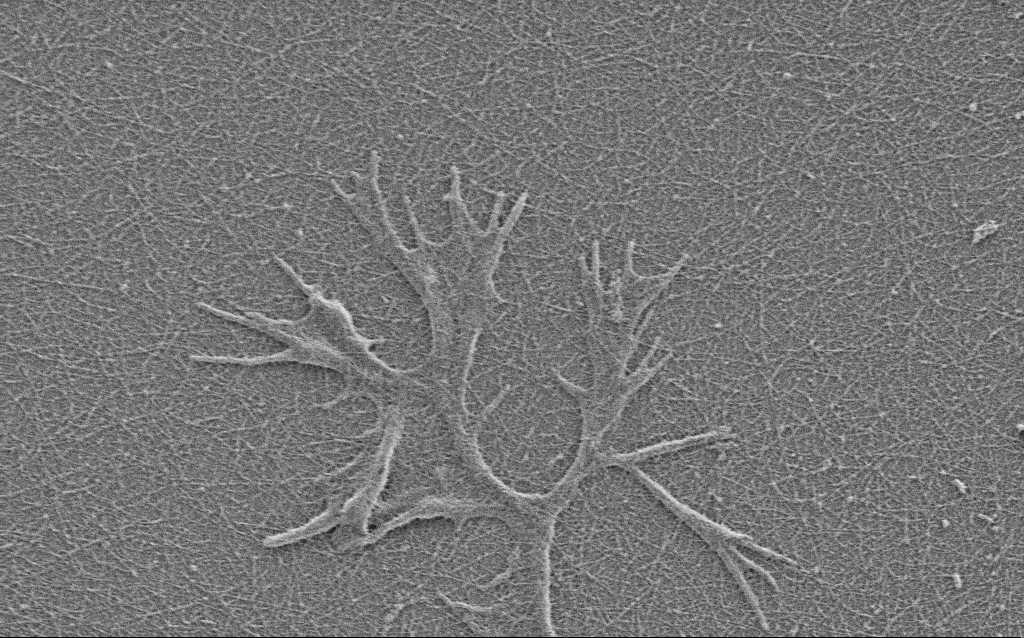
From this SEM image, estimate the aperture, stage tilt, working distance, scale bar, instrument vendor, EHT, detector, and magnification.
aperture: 30 µm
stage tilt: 0°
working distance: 4 mm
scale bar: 2000 nm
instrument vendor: Zeiss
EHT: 0.9 kV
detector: SE2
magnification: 15 K X